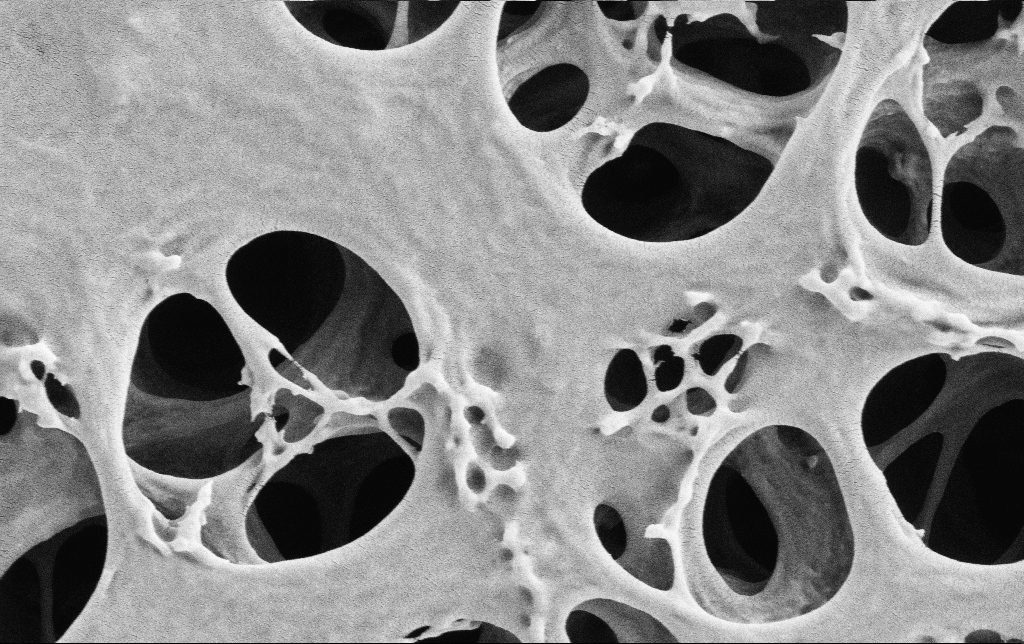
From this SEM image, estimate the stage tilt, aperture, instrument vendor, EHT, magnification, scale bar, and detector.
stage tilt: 0°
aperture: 30 µm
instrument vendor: Zeiss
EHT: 2 kV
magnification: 50 K X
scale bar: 1000 nm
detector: SE2